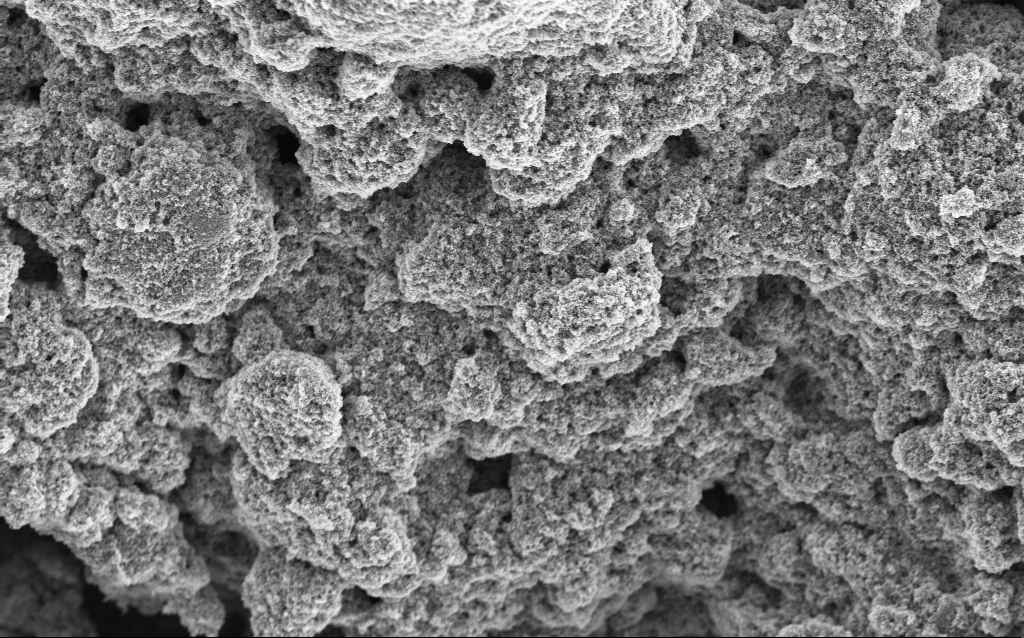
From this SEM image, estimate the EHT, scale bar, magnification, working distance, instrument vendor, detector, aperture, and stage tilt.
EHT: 5 kV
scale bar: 10000 nm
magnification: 6.4 K X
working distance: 4.5 mm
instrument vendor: Zeiss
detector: InLens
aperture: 30 µm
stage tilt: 0°